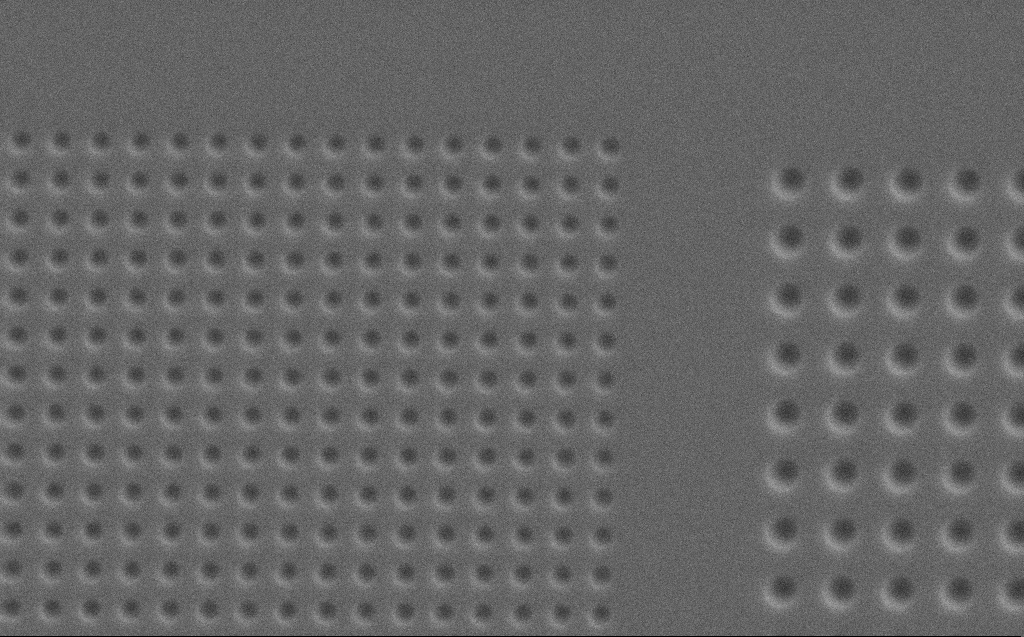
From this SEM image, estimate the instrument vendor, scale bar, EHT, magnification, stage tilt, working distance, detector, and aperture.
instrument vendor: Zeiss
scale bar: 2000 nm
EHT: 5 kV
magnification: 12.11 K X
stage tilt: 0°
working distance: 4 mm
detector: SE2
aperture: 30 µm